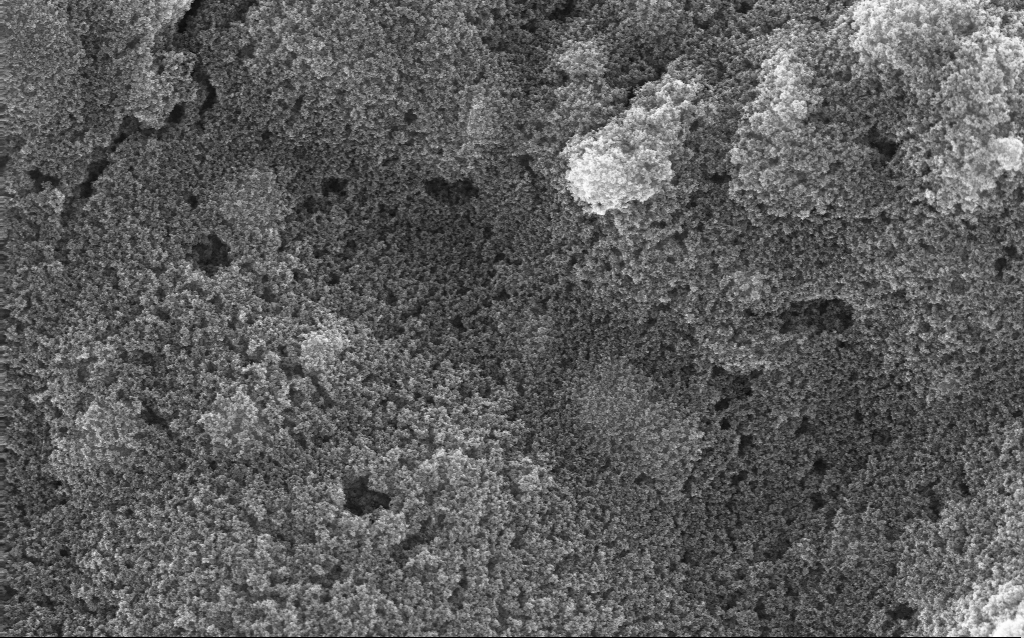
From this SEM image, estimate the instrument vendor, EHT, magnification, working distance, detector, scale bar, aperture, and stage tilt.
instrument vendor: Zeiss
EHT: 10 kV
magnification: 23.9 K X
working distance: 2.6 mm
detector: InLens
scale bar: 1000 nm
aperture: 30 µm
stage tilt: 0°